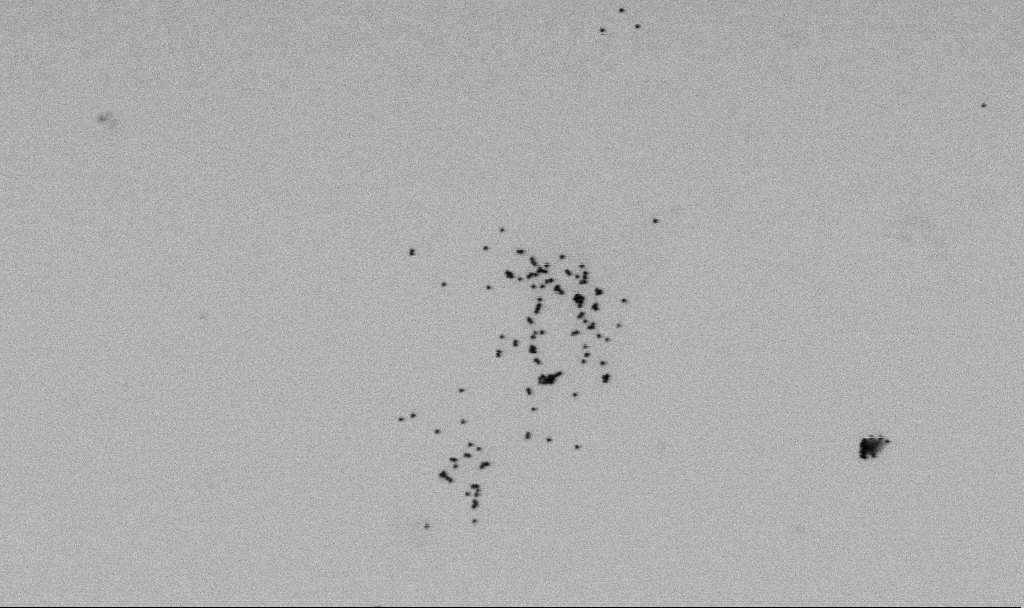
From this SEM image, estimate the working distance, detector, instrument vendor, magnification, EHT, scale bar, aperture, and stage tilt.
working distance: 6.5 mm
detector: SE2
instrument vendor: Zeiss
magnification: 60 K X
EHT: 2 kV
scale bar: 1000 nm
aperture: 30 µm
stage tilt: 0°